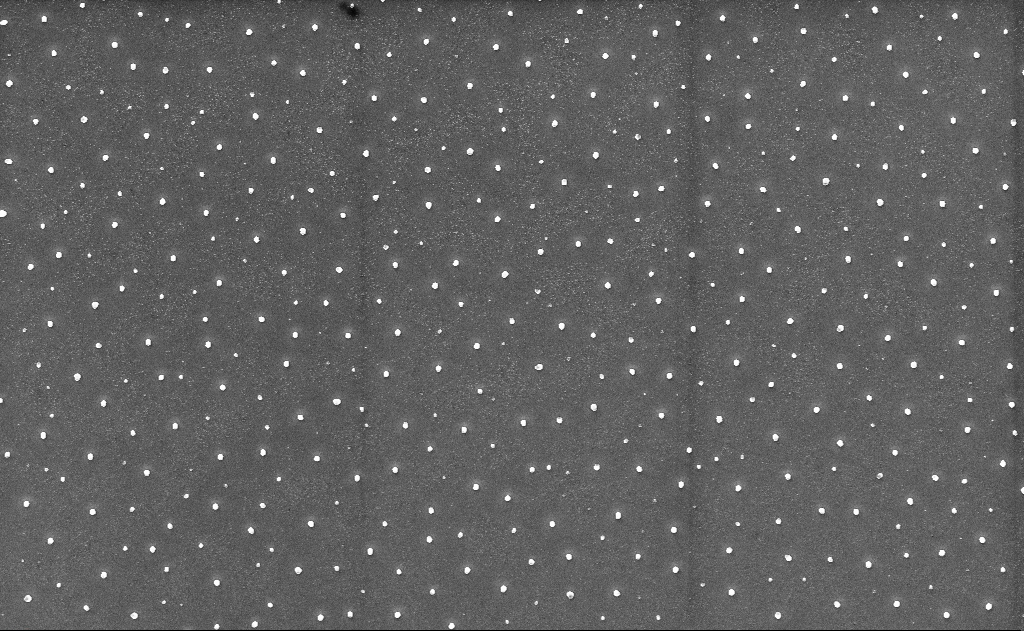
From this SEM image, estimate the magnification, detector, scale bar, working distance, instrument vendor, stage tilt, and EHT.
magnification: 5 K X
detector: InLens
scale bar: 10000 nm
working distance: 10 mm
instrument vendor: Zeiss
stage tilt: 0°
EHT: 10 kV